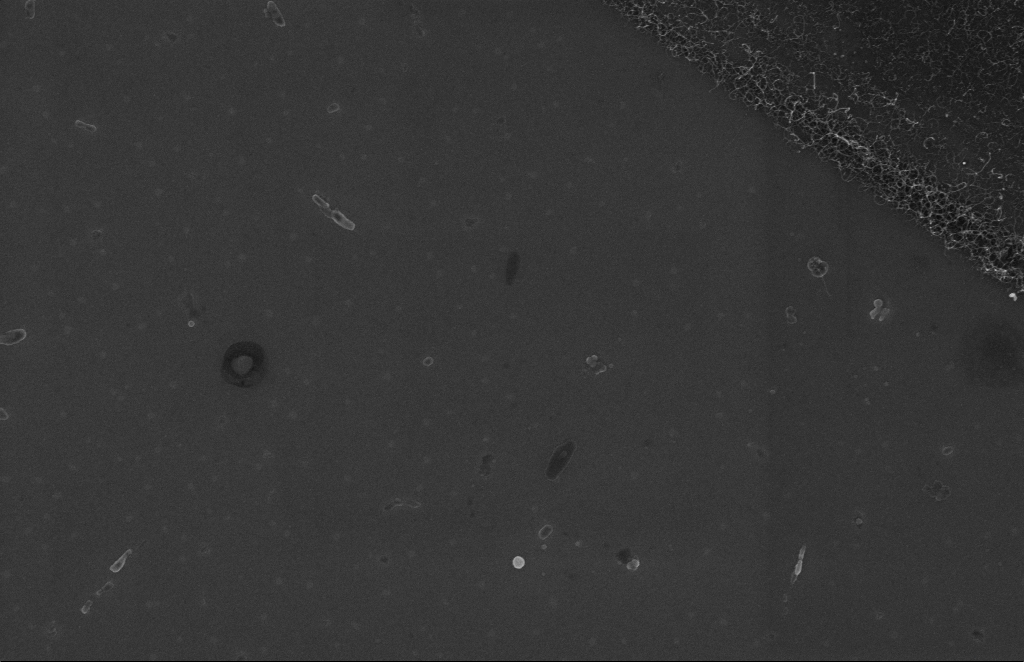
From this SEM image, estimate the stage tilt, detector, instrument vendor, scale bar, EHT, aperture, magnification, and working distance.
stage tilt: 0°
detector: InLens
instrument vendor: Zeiss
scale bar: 1000 nm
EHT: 5 kV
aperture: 20 µm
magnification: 31.32 K X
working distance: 5 mm